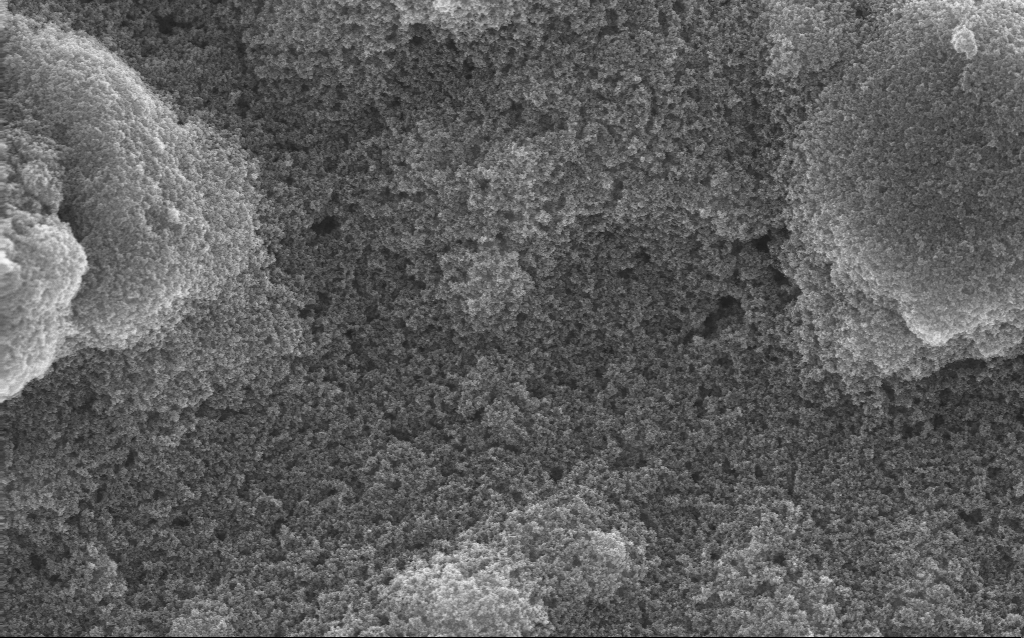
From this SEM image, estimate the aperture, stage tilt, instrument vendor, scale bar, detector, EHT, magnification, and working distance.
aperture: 30 µm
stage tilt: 0°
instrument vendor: Zeiss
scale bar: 1000 nm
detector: InLens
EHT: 10 kV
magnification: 23.9 K X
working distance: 2.7 mm